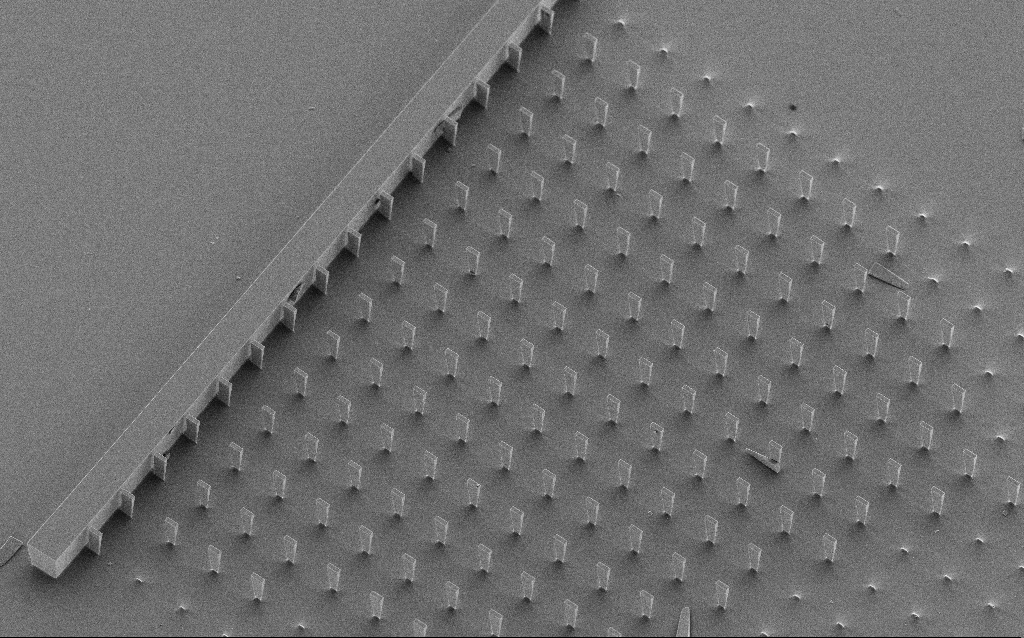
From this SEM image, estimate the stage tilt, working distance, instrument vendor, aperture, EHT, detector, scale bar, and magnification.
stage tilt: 30°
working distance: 10.1 mm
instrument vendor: Zeiss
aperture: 30 µm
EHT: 5 kV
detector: SE2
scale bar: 100000 nm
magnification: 0.663 K X